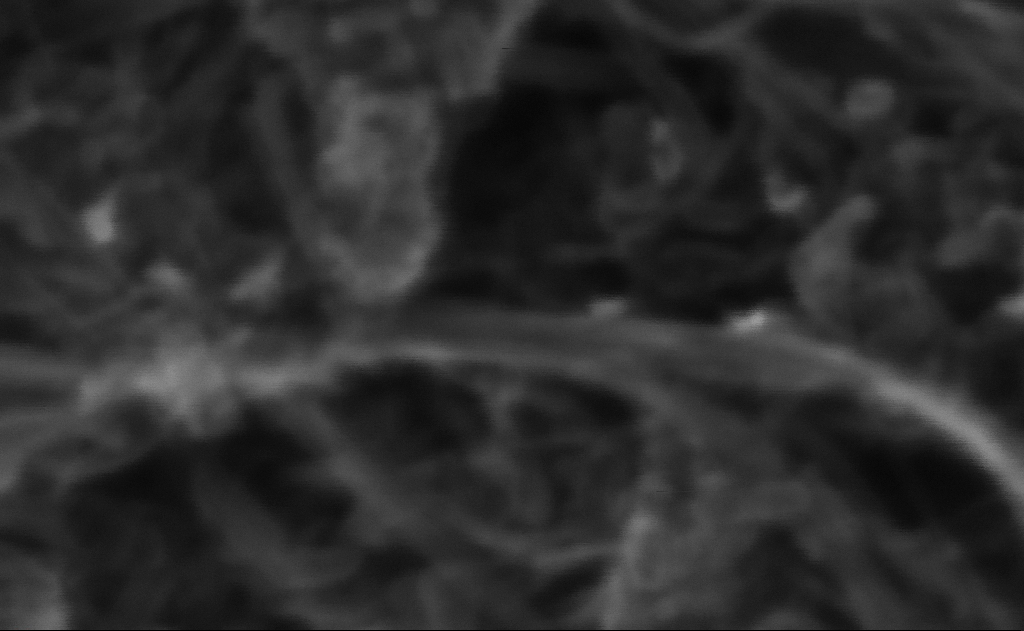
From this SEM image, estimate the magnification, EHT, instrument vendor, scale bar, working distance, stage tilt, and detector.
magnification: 1631.89 K X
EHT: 10 kV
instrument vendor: Zeiss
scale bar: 20 nm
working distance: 3 mm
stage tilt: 0°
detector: InLens